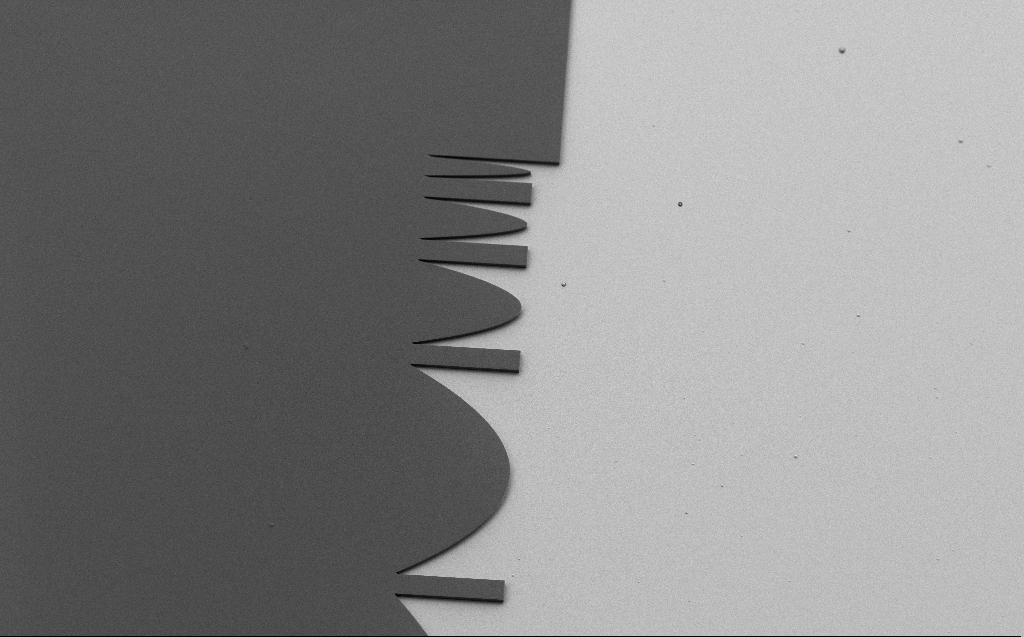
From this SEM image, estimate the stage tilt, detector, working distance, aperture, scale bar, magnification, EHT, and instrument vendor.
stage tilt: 30°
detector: SE2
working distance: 6 mm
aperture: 30 µm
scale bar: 20000 nm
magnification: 0.787 K X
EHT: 1.1 kV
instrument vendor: Zeiss